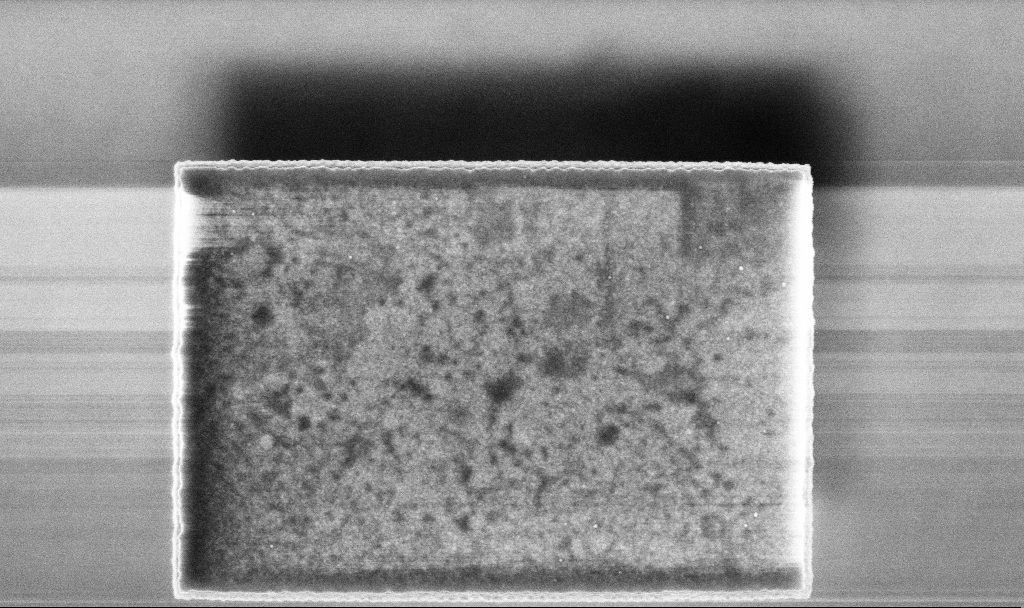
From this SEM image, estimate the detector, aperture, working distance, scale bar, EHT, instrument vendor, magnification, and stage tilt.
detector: InLens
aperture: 30 µm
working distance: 3.3 mm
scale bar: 1000 nm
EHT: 5 kV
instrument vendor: Zeiss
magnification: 52.64 K X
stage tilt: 0°